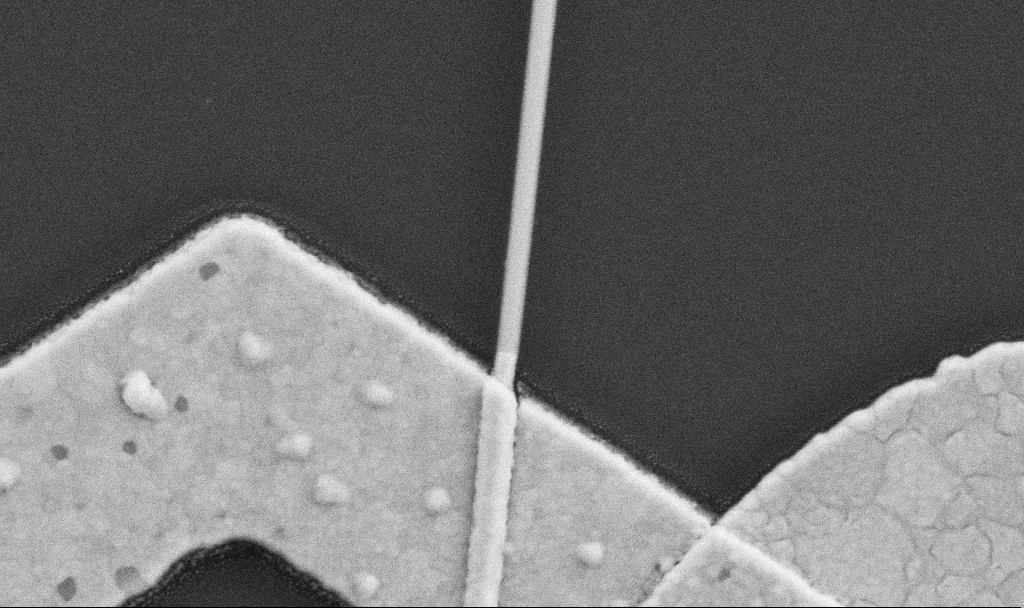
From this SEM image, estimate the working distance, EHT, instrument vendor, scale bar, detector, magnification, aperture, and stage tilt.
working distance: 8.7 mm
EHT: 5 kV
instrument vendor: Zeiss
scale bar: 200 nm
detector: SE2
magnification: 100 K X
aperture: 30 µm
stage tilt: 0°